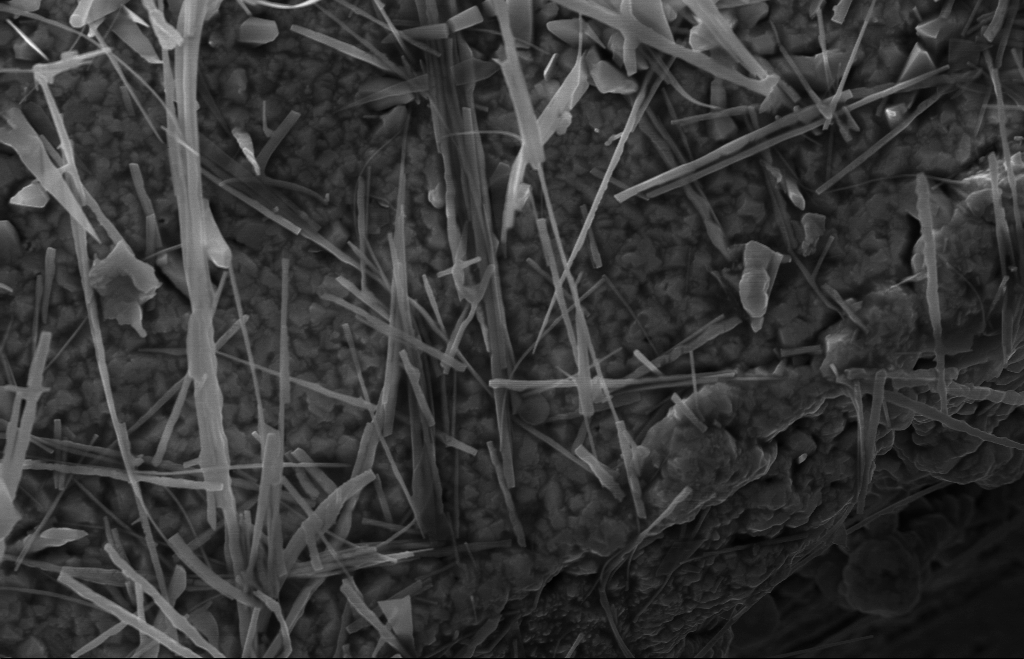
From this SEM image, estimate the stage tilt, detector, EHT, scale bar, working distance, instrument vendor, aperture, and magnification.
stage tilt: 0°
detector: InLens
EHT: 10 kV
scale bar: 2000 nm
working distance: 9 mm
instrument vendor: Zeiss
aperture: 30 µm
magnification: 20 K X